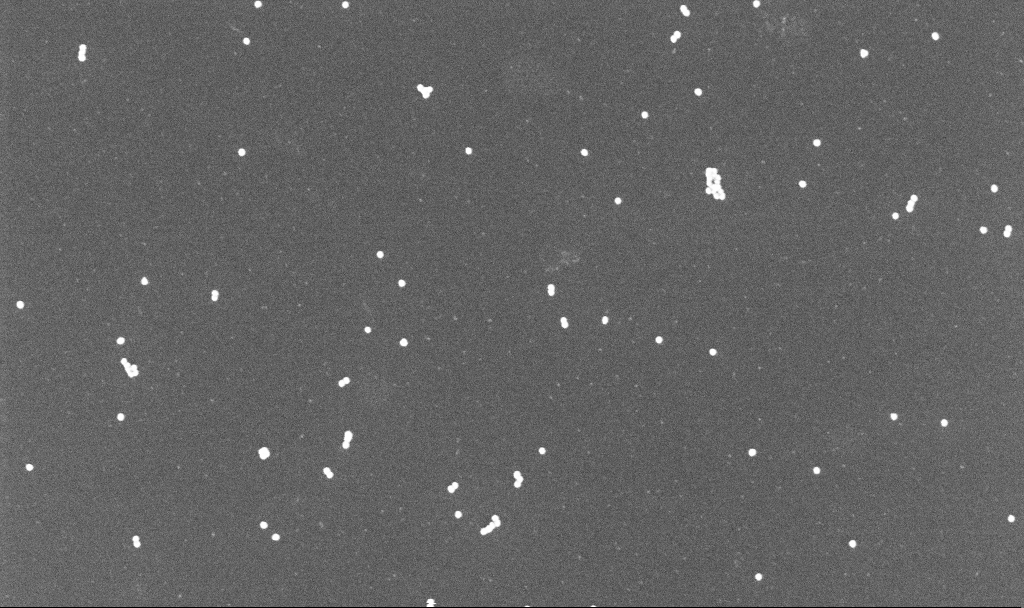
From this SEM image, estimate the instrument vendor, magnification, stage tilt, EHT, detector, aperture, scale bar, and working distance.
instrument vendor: Zeiss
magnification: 100 K X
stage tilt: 0°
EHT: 10 kV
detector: InLens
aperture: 30 µm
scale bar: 200 nm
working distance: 3.3 mm